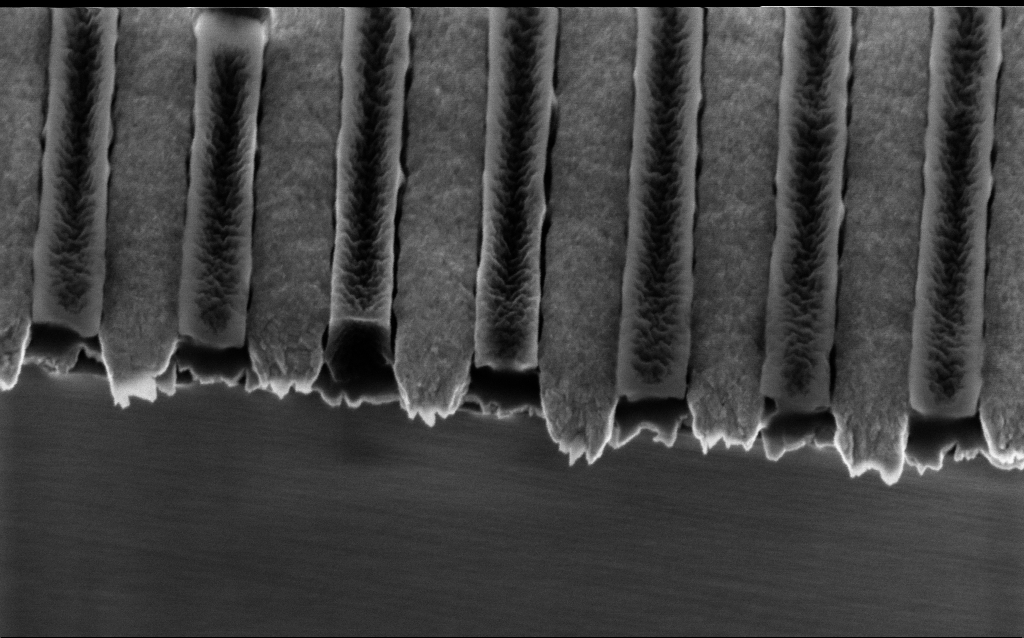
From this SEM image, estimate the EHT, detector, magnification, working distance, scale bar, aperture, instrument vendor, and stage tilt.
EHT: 2 kV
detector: InLens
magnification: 108.32 K X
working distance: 4.2 mm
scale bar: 200 nm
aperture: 30 µm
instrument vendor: Zeiss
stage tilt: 32°